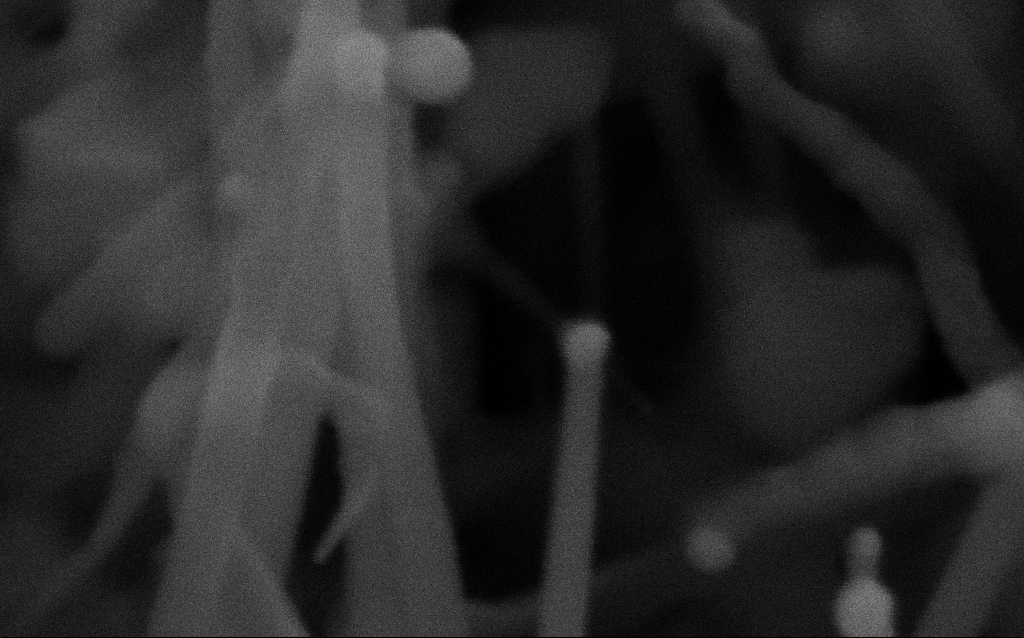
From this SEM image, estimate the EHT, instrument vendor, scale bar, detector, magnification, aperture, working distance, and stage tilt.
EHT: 10 kV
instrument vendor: Zeiss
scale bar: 100 nm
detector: SE2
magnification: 678.82 K X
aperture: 30 µm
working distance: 7.3 mm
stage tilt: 35°